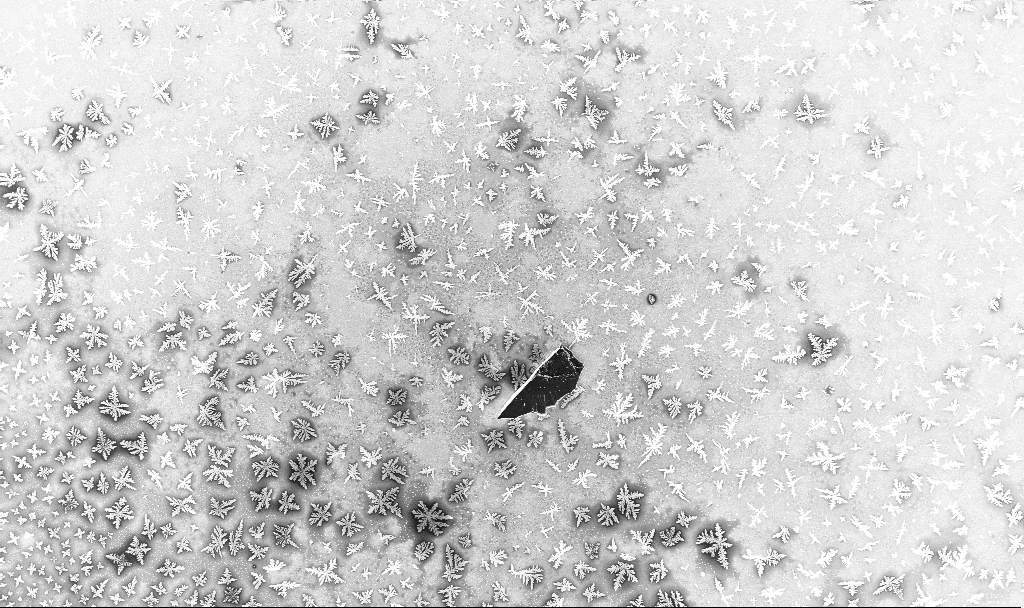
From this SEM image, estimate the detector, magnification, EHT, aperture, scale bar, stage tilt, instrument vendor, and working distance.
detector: InLens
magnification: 0.436 K X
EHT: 5 kV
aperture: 30 µm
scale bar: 100000 nm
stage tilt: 0°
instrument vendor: Zeiss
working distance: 3.3 mm